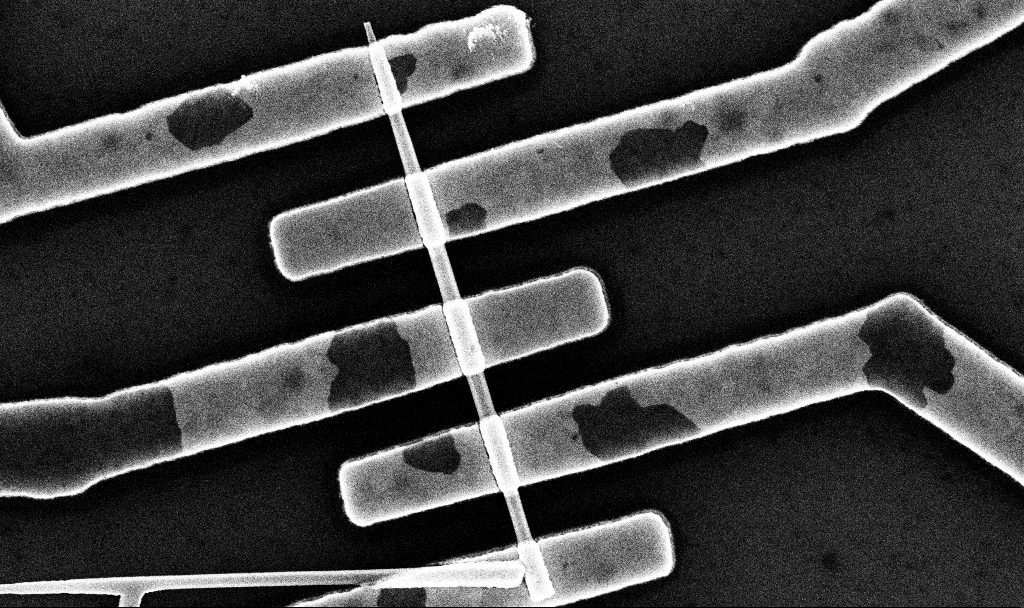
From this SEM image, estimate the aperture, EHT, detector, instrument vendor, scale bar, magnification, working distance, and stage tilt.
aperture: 30 µm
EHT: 10 kV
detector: InLens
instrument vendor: Zeiss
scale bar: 1000 nm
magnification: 43.07 K X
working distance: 6.7 mm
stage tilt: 0°